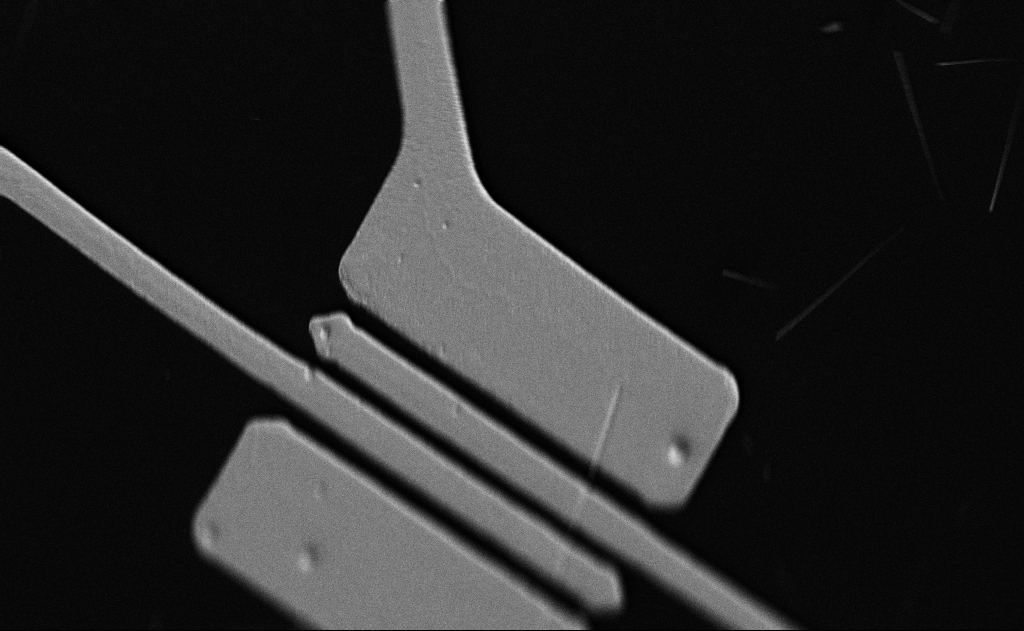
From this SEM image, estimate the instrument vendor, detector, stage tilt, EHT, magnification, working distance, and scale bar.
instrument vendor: Zeiss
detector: SE2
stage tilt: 0°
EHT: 5 kV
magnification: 5 K X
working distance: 21 mm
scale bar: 10000 nm